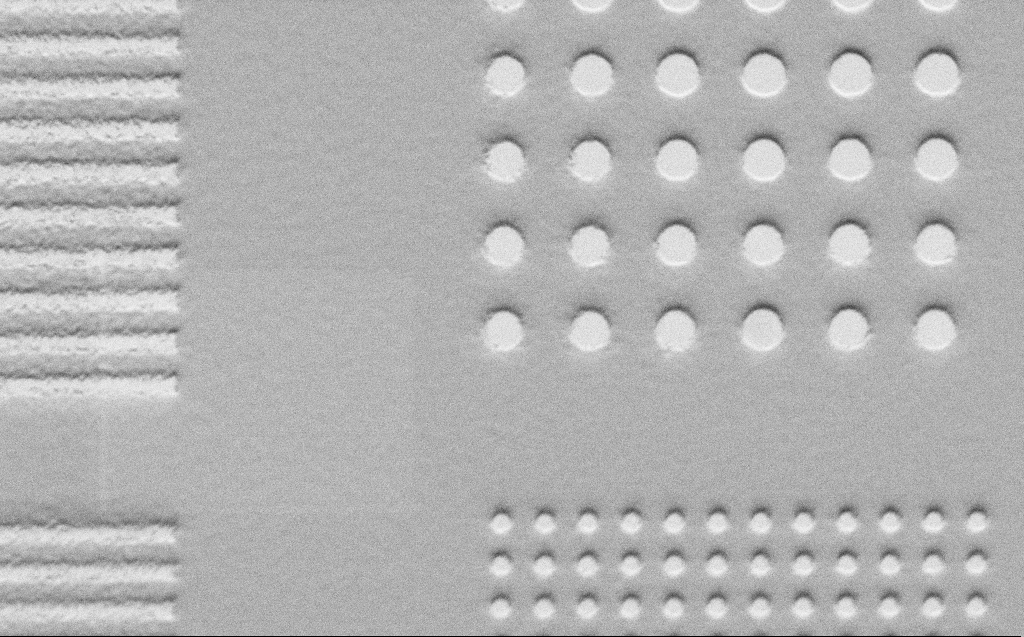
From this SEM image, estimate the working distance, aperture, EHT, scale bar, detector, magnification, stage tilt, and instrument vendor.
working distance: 5 mm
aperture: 30 µm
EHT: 1 kV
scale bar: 2000 nm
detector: SE2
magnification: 8.06 K X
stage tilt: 45°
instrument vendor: Zeiss